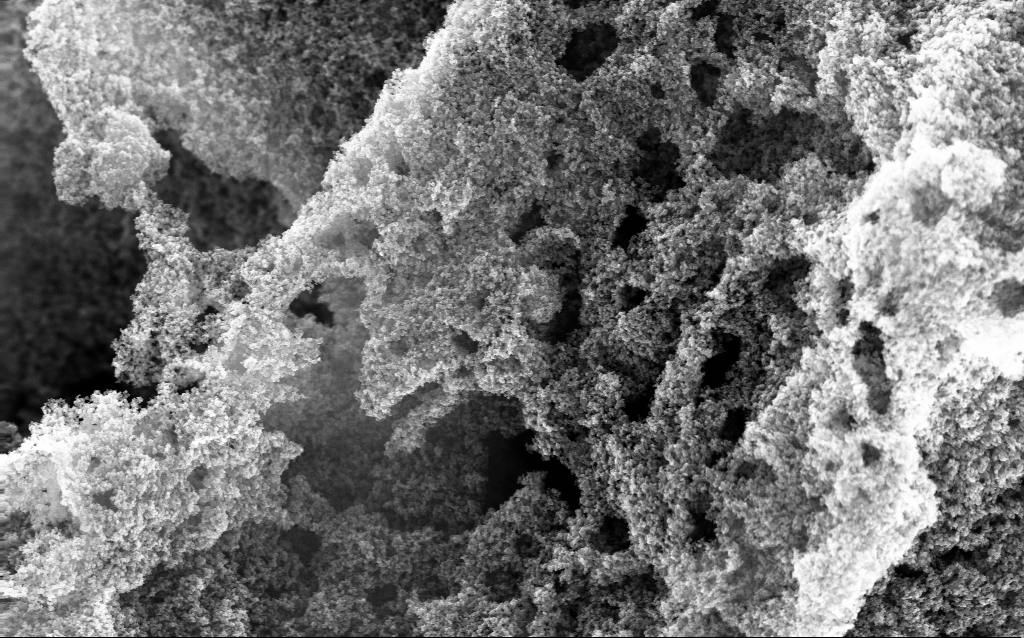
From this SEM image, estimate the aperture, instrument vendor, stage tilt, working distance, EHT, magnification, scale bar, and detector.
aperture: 30 µm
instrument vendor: Zeiss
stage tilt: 0°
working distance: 2.4 mm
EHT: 10 kV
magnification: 37.88 K X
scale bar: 1000 nm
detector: InLens